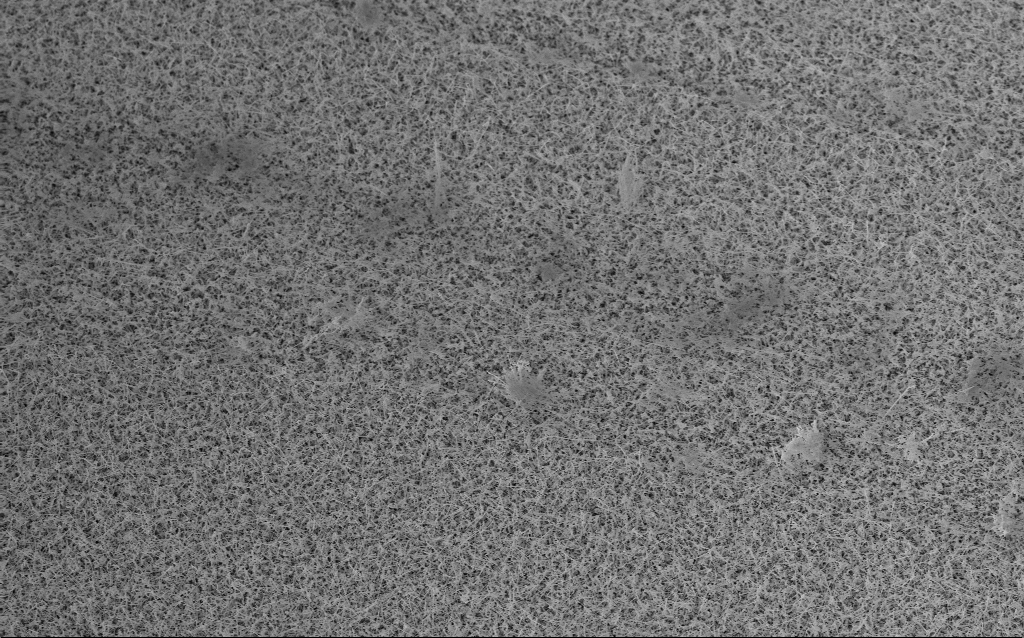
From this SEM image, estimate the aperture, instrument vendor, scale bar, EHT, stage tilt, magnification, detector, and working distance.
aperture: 30 µm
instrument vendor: Zeiss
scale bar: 2000 nm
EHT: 5 kV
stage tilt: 20°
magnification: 10 K X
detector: InLens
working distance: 6.4 mm